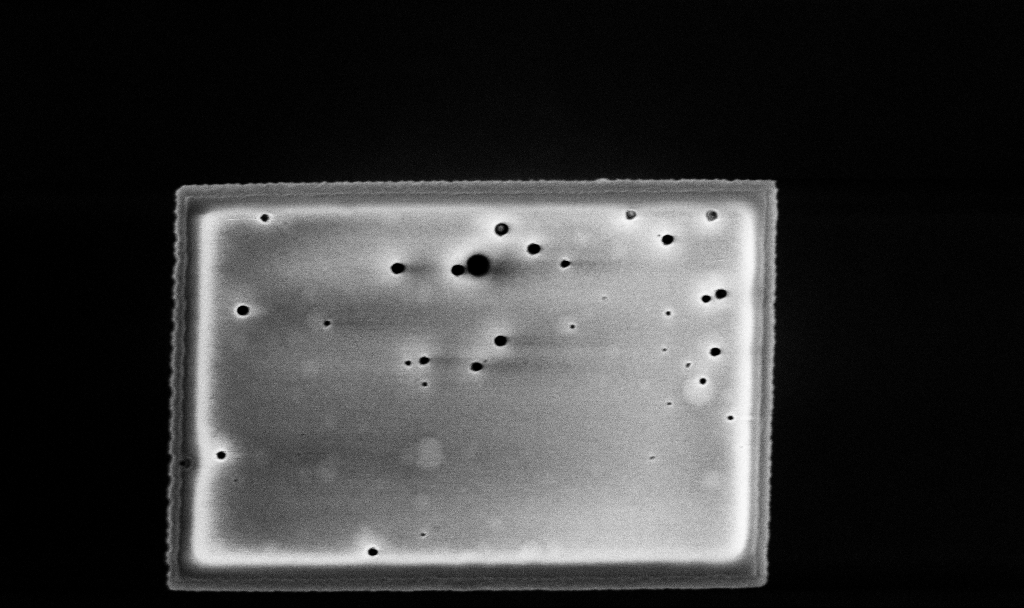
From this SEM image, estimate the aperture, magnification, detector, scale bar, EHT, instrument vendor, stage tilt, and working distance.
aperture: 30 µm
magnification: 51.38 K X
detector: InLens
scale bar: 1000 nm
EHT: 5 kV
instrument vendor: Zeiss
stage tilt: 0°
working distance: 3.3 mm